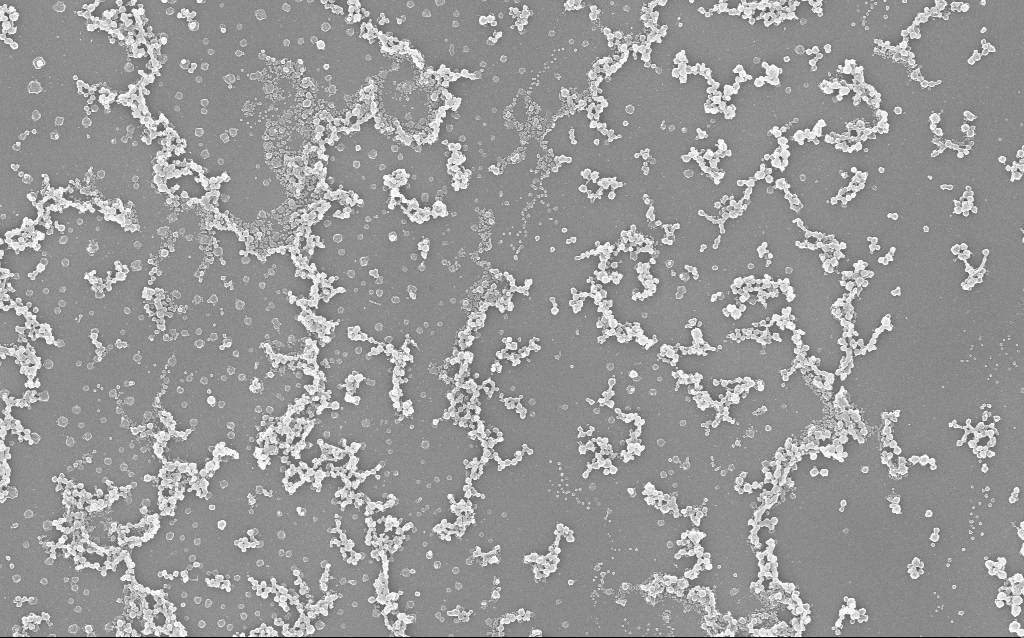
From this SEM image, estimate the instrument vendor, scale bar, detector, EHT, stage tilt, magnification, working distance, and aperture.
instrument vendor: Zeiss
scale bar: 2000 nm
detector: InLens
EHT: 20 kV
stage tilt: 0°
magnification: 10 K X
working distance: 1.7 mm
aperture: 30 µm